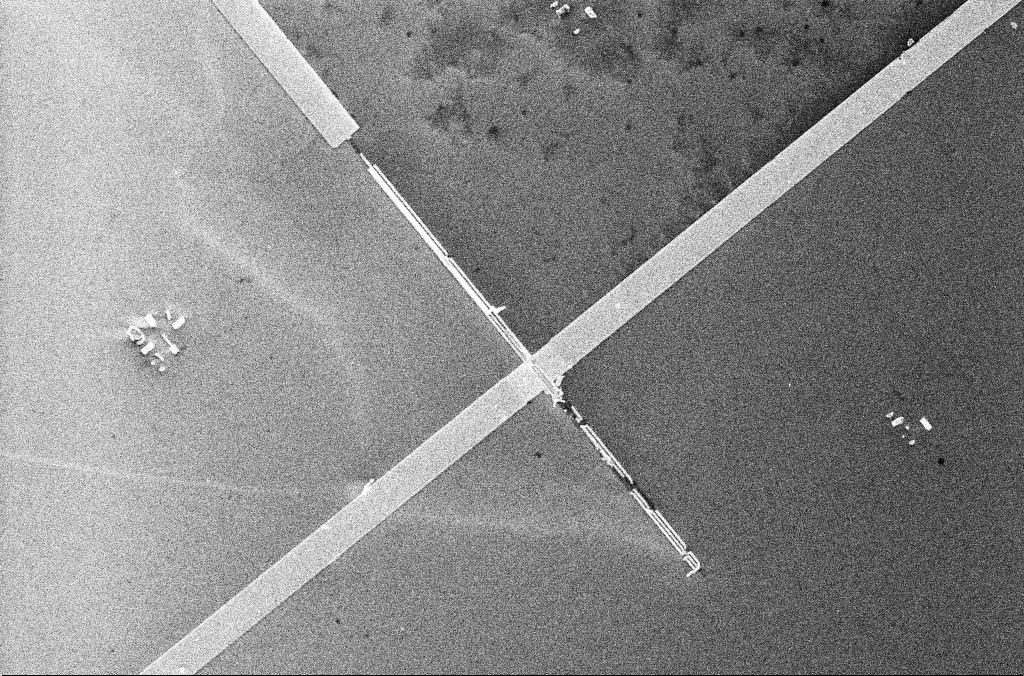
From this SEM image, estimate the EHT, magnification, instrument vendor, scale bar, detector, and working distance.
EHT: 5 kV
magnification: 2.47 K X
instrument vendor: Zeiss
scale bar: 20000 nm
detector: InLens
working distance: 3.4 mm